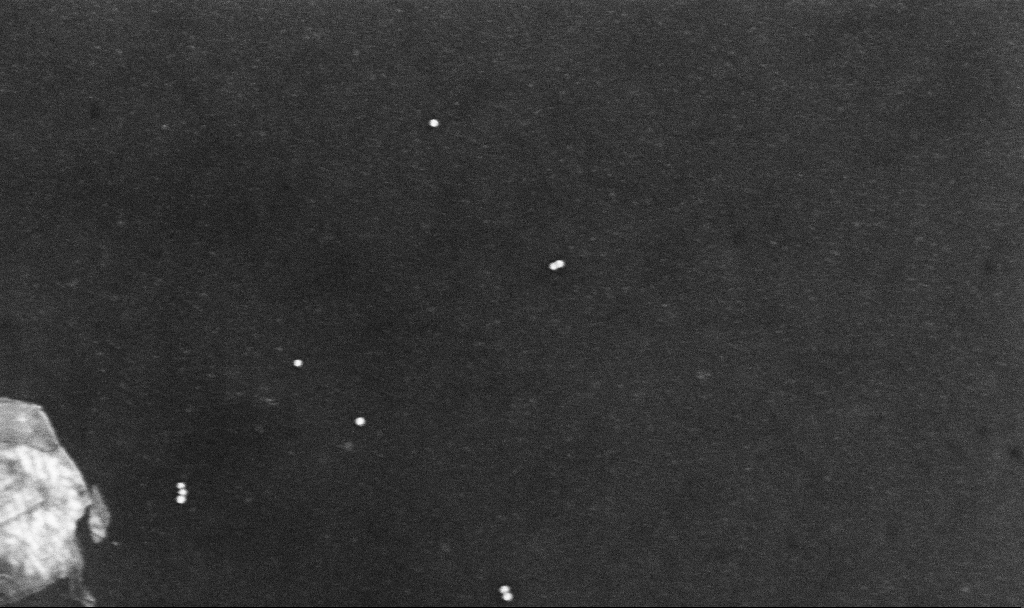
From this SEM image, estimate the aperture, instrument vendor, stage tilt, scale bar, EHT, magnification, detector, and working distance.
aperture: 30 µm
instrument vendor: Zeiss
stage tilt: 0°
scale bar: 200 nm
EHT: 10 kV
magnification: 118.19 K X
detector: InLens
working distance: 3.3 mm